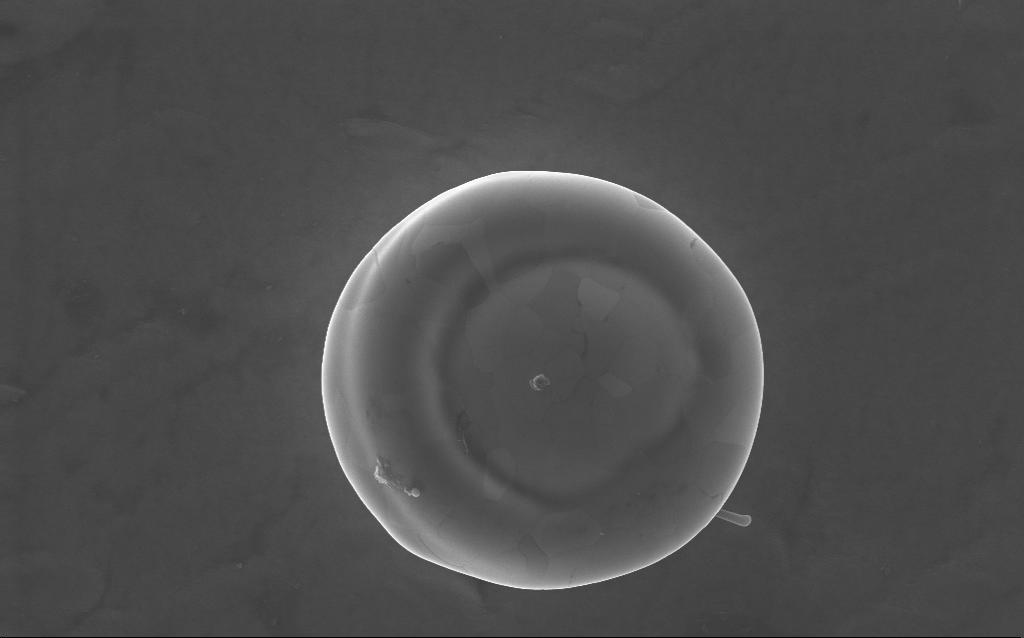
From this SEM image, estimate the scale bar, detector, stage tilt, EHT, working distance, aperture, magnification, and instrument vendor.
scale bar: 2000 nm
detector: InLens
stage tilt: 0°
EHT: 10 kV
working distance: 2 mm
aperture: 30 µm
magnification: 36 K X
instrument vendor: Zeiss